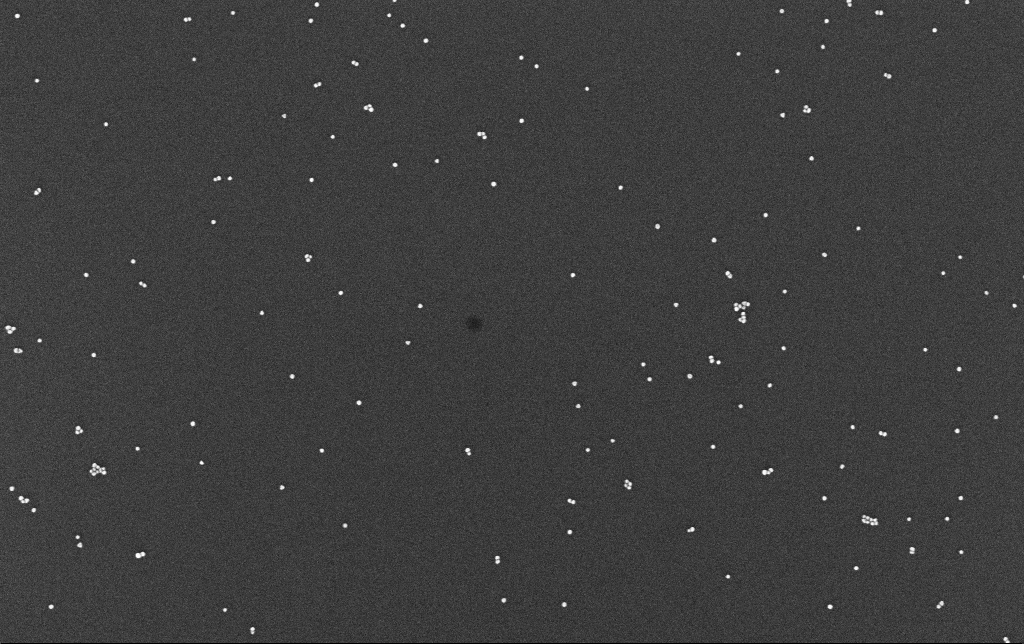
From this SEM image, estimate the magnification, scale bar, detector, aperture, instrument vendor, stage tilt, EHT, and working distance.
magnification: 100 K X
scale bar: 200 nm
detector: InLens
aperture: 30 µm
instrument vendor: Zeiss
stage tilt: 0°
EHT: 10 kV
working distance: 3.3 mm